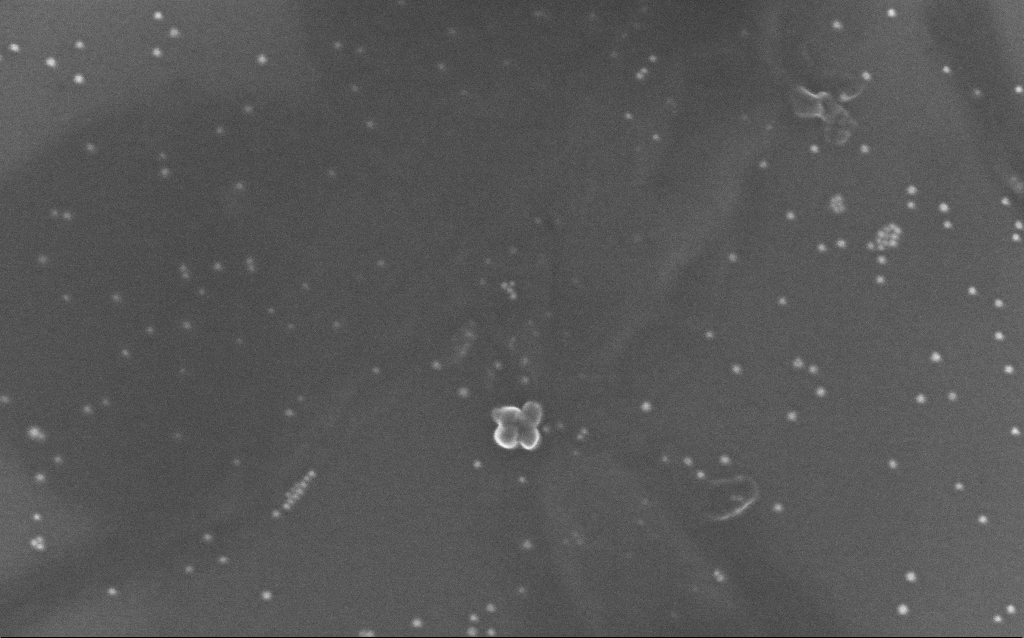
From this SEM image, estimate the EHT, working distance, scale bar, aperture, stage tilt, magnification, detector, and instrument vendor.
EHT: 10 kV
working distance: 6.6 mm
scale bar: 200 nm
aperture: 30 µm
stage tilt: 0°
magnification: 172.44 K X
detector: InLens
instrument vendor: Zeiss